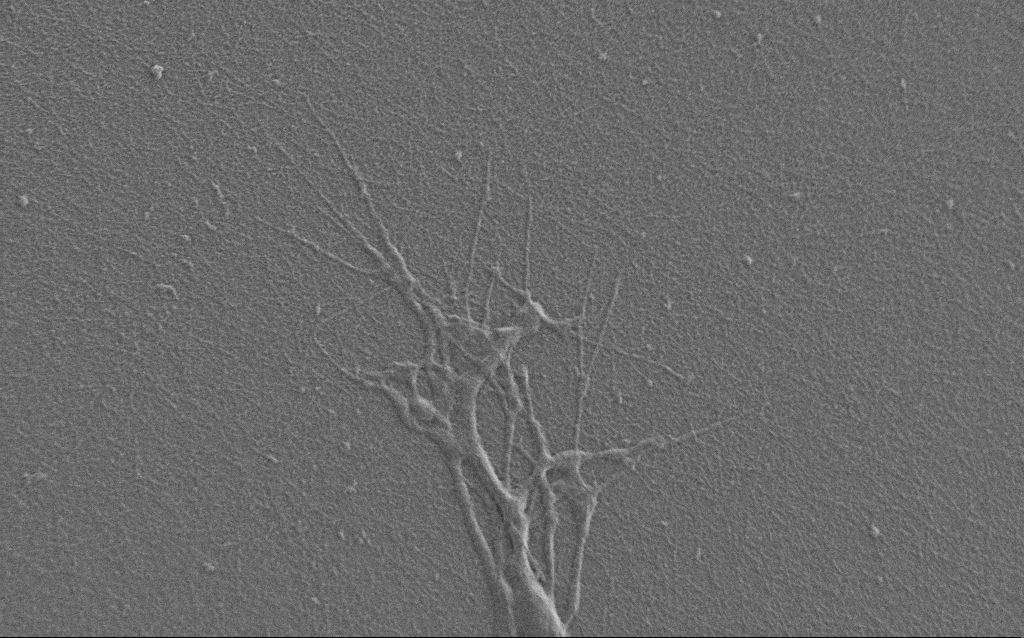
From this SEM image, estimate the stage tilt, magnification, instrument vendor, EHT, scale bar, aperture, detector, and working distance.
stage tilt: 0°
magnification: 7 K X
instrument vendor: Zeiss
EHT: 0.9 kV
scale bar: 10000 nm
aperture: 30 µm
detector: SE2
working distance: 7 mm